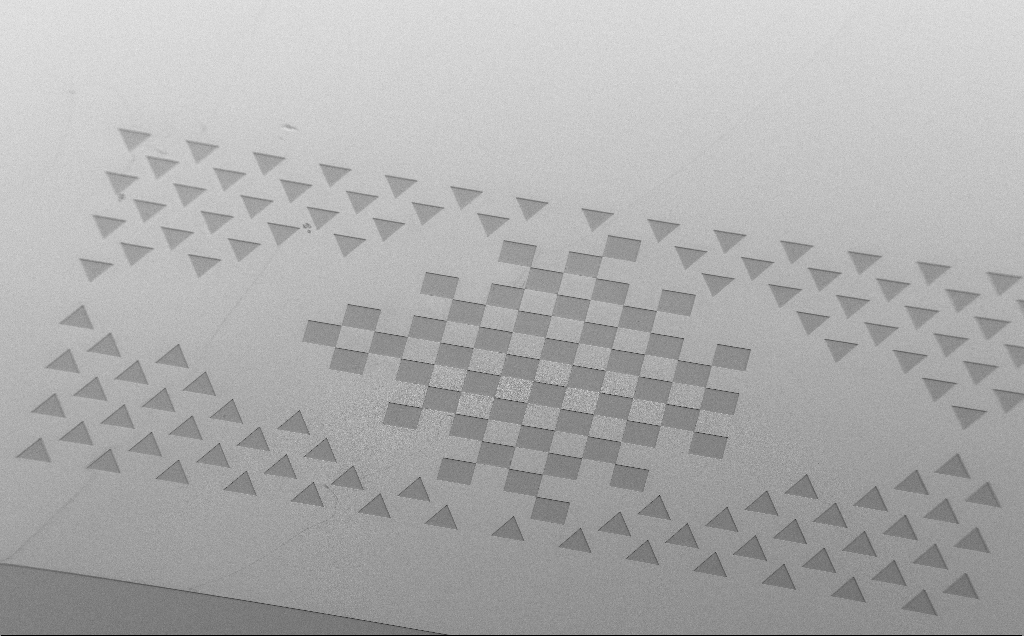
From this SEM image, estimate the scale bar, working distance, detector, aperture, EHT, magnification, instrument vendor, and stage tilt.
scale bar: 200000 nm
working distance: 13 mm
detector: SE2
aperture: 30 µm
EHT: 5 kV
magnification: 0.108 K X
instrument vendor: Zeiss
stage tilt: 35°